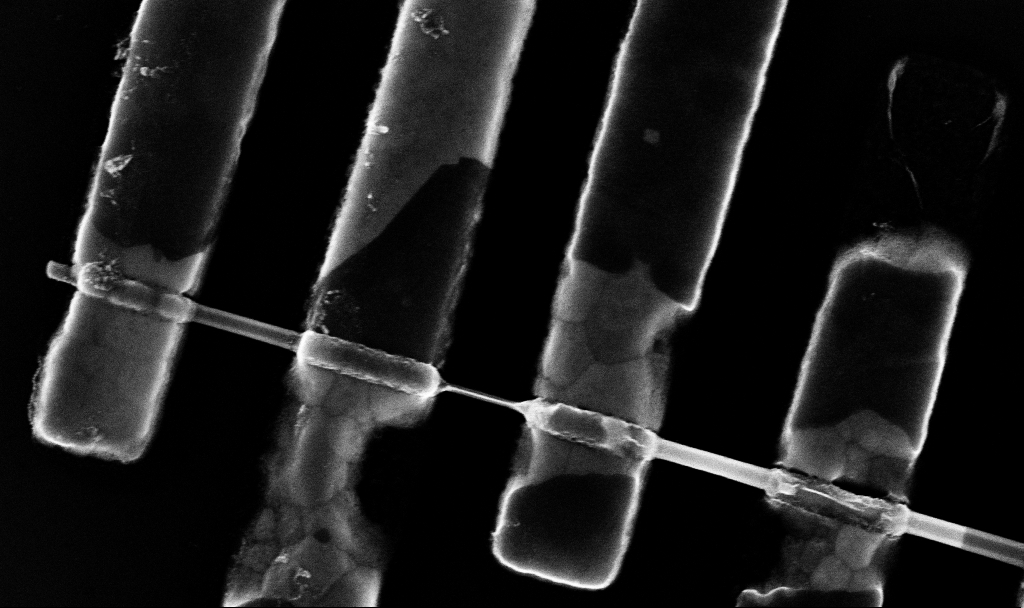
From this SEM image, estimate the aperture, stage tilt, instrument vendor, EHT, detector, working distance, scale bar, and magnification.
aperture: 30 µm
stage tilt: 0°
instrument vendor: Zeiss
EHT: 10 kV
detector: InLens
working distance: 6.8 mm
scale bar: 200 nm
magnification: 82.65 K X